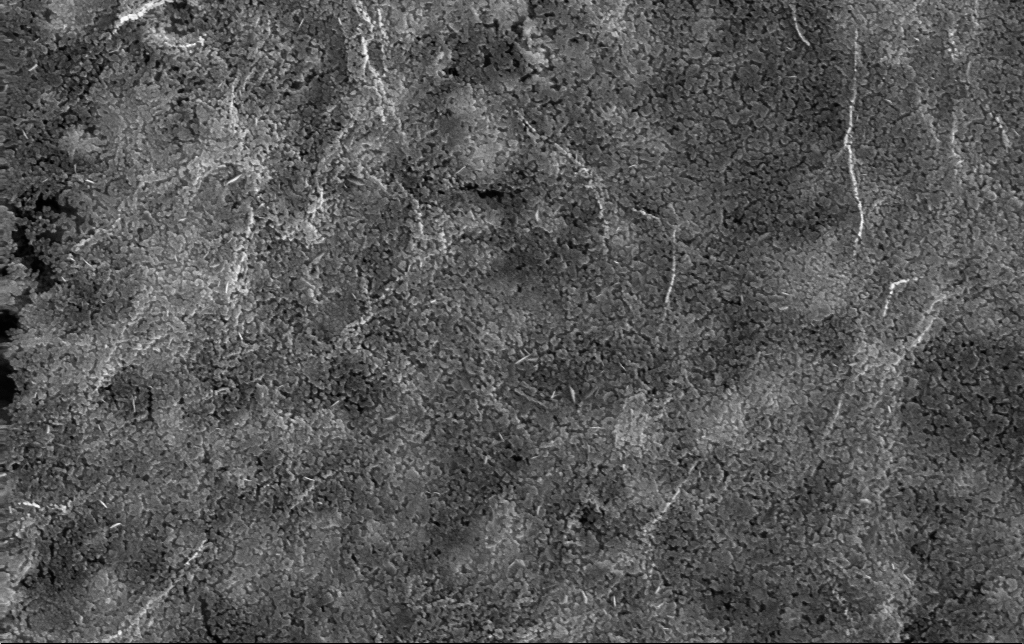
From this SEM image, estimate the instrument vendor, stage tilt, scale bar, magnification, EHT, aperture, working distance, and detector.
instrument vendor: Zeiss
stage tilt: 0°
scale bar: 200 nm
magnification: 80 K X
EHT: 10 kV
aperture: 30 µm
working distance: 3 mm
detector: InLens